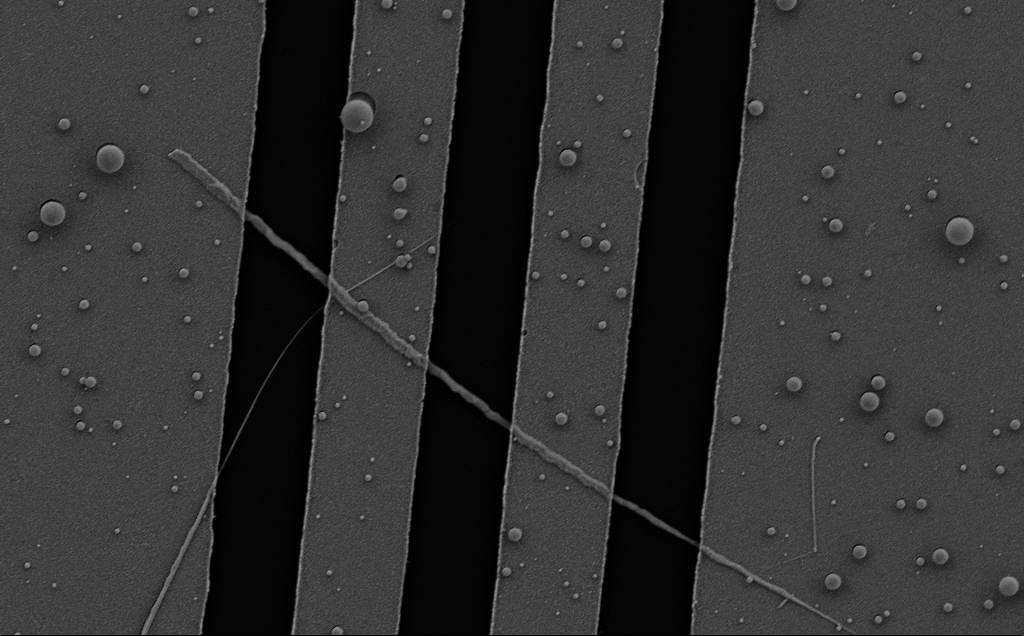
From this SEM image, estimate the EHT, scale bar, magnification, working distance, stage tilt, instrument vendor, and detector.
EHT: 5 kV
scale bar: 2000 nm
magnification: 18.24 K X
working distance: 8 mm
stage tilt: -0.7°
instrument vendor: Zeiss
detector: SE2